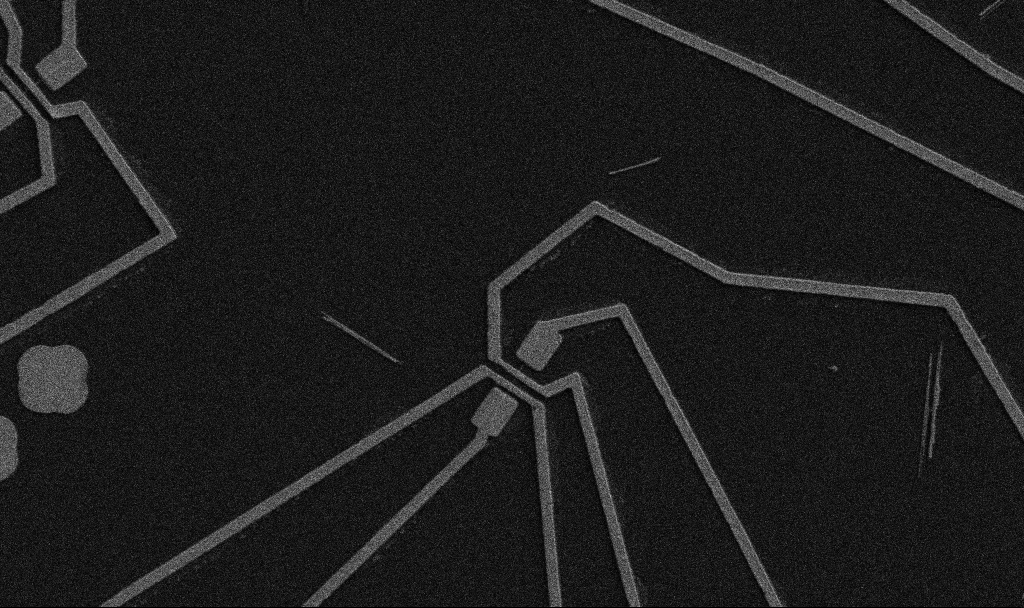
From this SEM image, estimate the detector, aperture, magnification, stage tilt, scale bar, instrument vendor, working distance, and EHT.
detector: SE2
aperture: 30 µm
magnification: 5 K X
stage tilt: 0°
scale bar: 10000 nm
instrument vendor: Zeiss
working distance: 10.7 mm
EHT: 5 kV